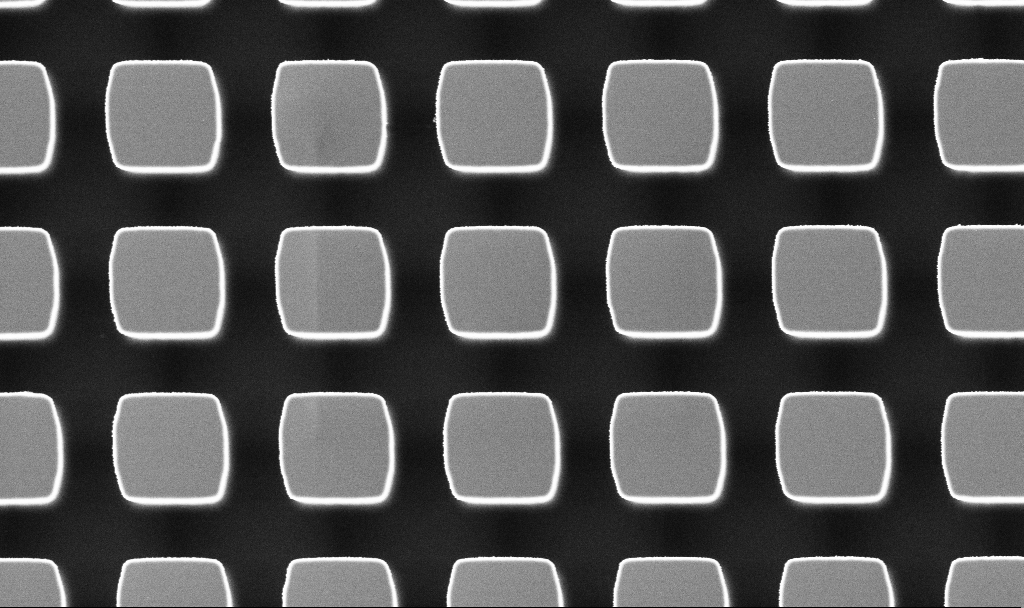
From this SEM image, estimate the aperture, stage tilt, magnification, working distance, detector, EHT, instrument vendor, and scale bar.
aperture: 30 µm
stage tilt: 0°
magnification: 10.25 K X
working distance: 3.2 mm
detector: InLens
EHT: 3 kV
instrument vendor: Zeiss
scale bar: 2000 nm